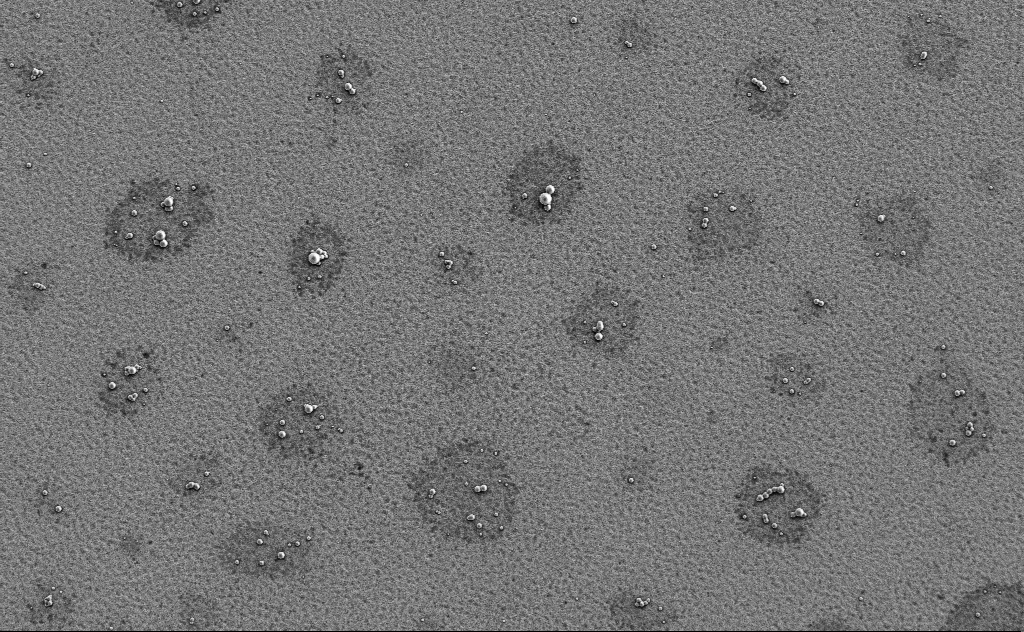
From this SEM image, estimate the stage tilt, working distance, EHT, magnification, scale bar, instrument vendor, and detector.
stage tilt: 0°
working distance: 13 mm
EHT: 3 kV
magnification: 2.07 K X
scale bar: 10000 nm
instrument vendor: Zeiss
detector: SE2